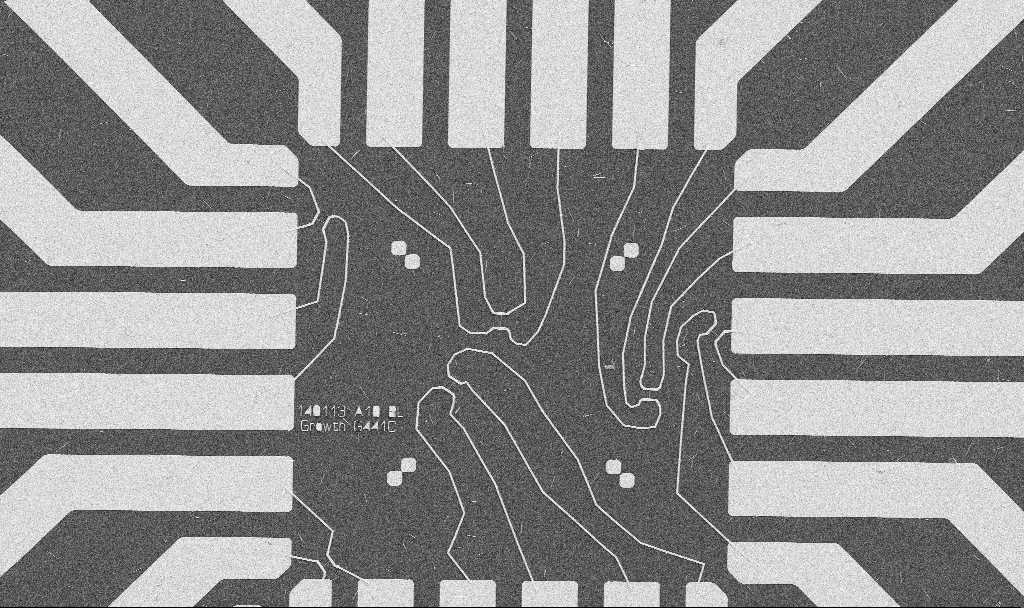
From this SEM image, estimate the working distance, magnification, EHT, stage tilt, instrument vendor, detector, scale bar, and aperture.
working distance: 10.7 mm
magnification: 1 K X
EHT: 5 kV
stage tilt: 0°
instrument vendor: Zeiss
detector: SE2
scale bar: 20000 nm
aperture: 30 µm